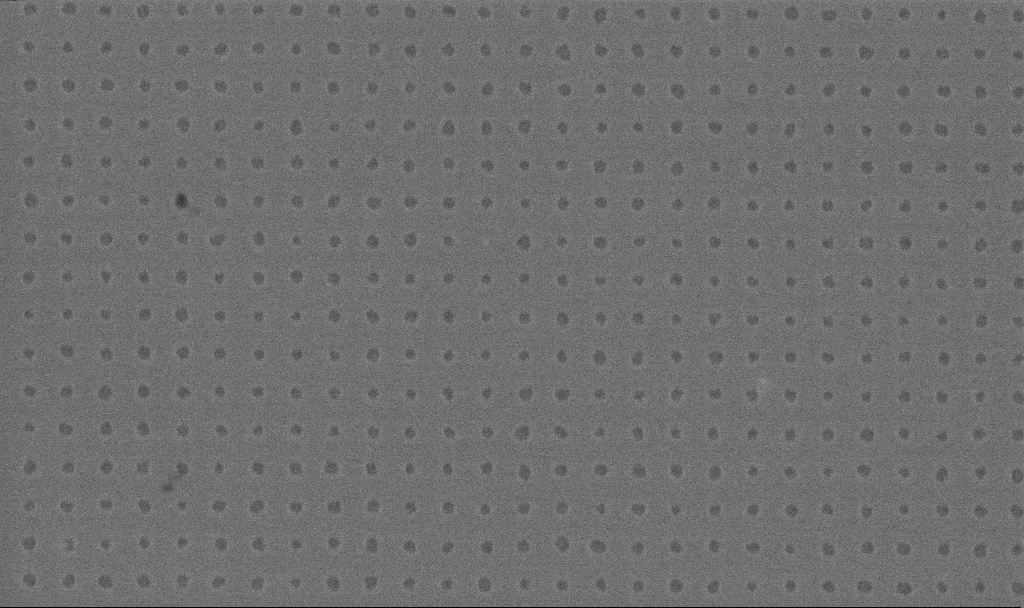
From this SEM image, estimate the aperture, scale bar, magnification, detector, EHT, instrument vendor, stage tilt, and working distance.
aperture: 30 µm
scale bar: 1000 nm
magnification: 46.88 K X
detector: InLens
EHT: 3 kV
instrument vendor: Zeiss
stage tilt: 0°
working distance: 3.5 mm